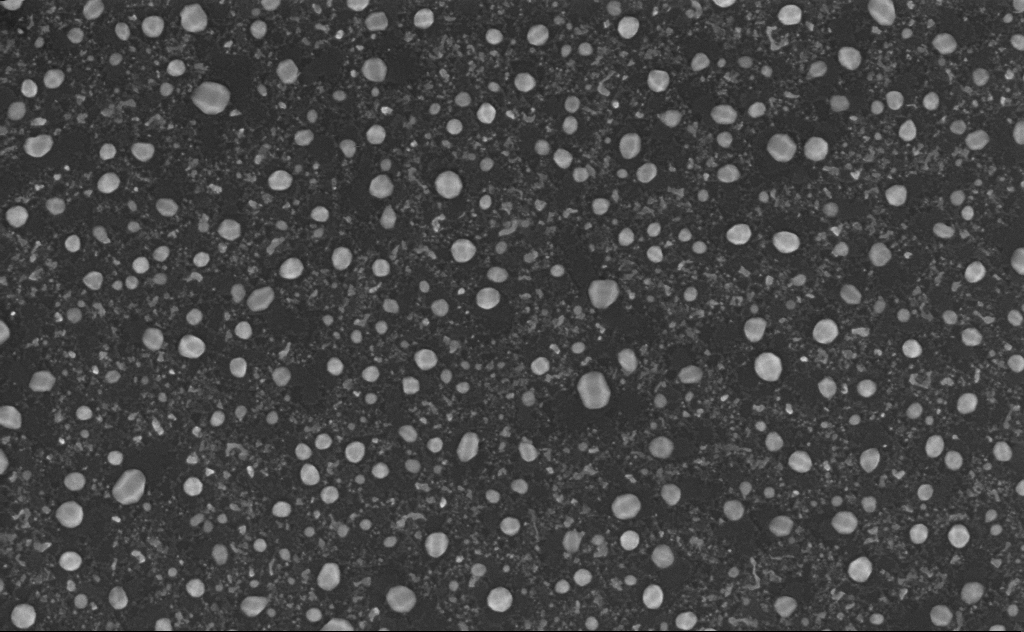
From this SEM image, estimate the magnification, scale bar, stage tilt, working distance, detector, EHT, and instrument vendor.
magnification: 80 K X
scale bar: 200 nm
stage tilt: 0°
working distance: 4 mm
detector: InLens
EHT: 5 kV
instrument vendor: Zeiss